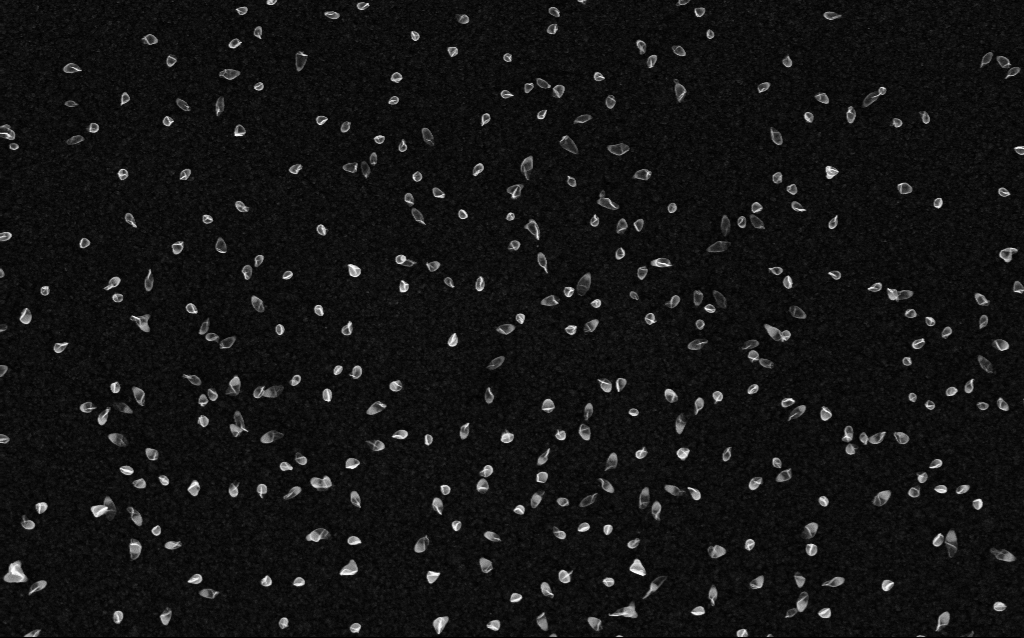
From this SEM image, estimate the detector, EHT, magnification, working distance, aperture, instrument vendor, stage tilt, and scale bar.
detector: InLens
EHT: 5 kV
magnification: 50 K X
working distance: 2.1 mm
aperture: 30 µm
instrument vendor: Zeiss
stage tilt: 0°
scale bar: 1000 nm